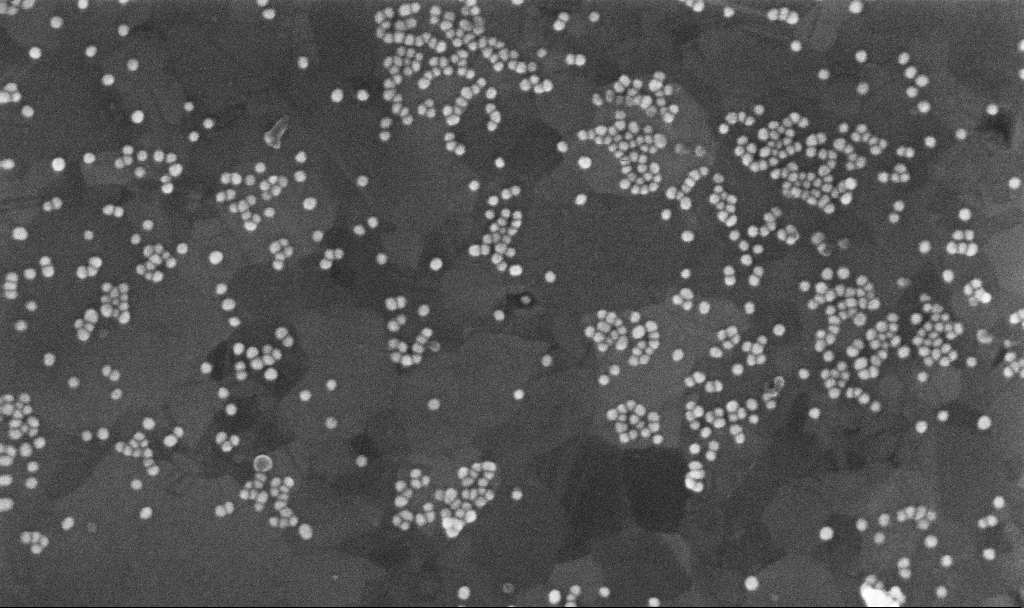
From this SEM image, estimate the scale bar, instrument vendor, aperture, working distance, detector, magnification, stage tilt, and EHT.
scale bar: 200 nm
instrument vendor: Zeiss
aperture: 30 µm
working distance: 3.7 mm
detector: InLens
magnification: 200 K X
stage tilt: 0°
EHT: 10 kV